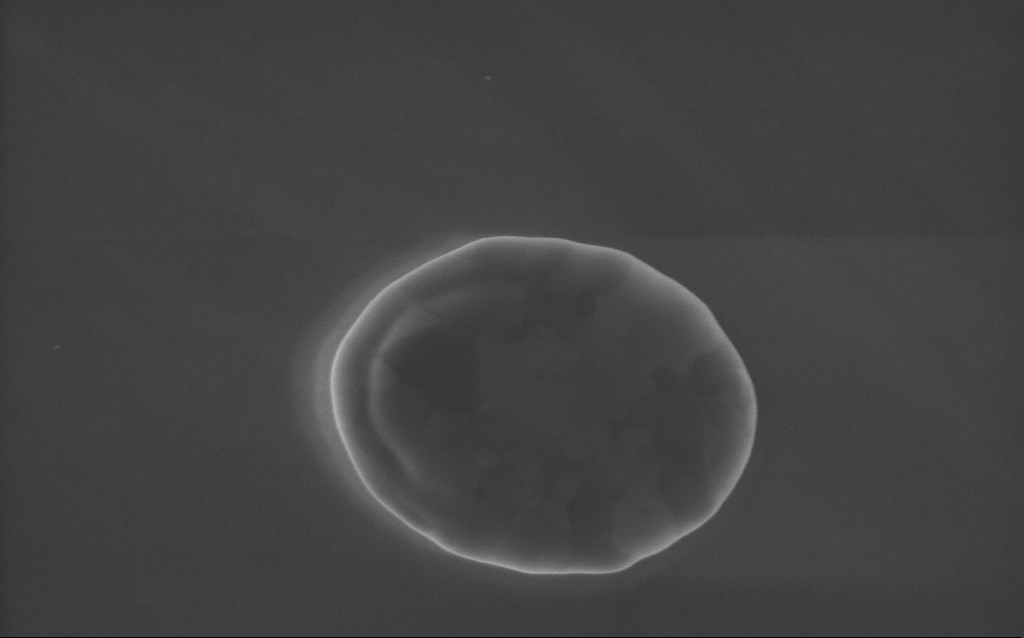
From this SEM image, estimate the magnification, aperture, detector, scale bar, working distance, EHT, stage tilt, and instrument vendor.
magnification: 53 K X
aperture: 30 µm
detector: InLens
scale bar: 1000 nm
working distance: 4 mm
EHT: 5 kV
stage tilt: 0°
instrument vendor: Zeiss